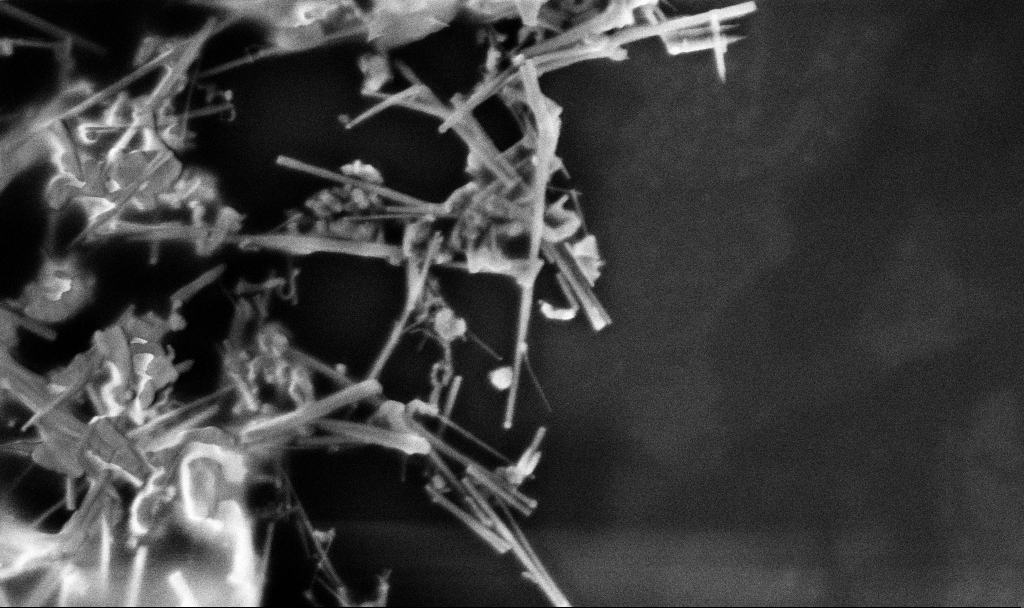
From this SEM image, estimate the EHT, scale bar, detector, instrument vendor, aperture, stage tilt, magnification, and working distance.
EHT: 3 kV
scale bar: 200 nm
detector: InLens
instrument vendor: Zeiss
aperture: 30 µm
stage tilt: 0°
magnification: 74.65 K X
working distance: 3.3 mm